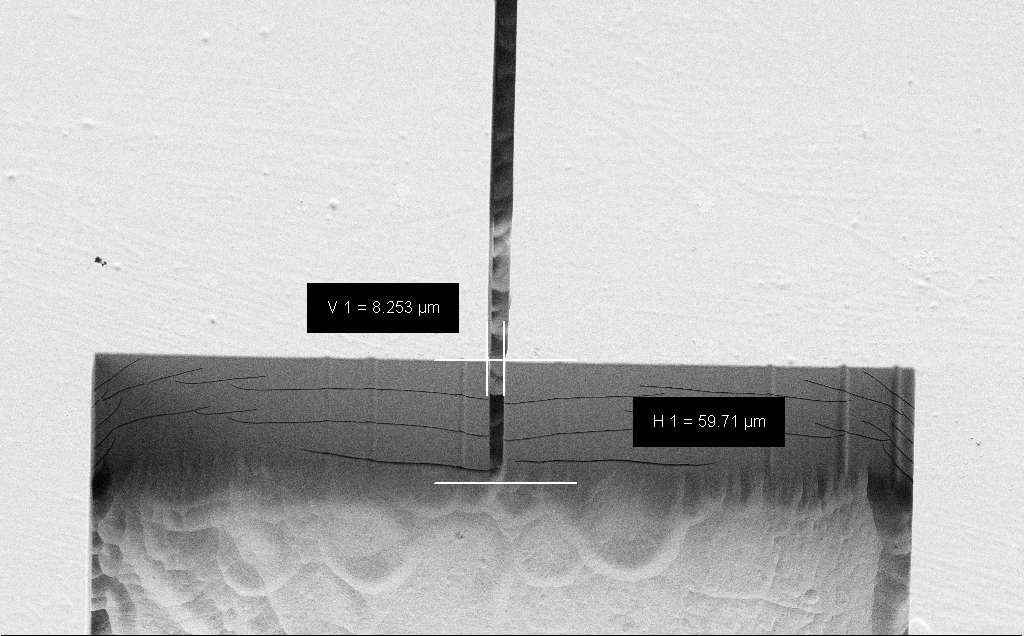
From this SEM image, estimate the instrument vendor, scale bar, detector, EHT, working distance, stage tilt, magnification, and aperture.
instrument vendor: Zeiss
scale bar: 20000 nm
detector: SE2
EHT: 1.2 kV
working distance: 5 mm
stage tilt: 45°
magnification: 0.756 K X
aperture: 30 µm